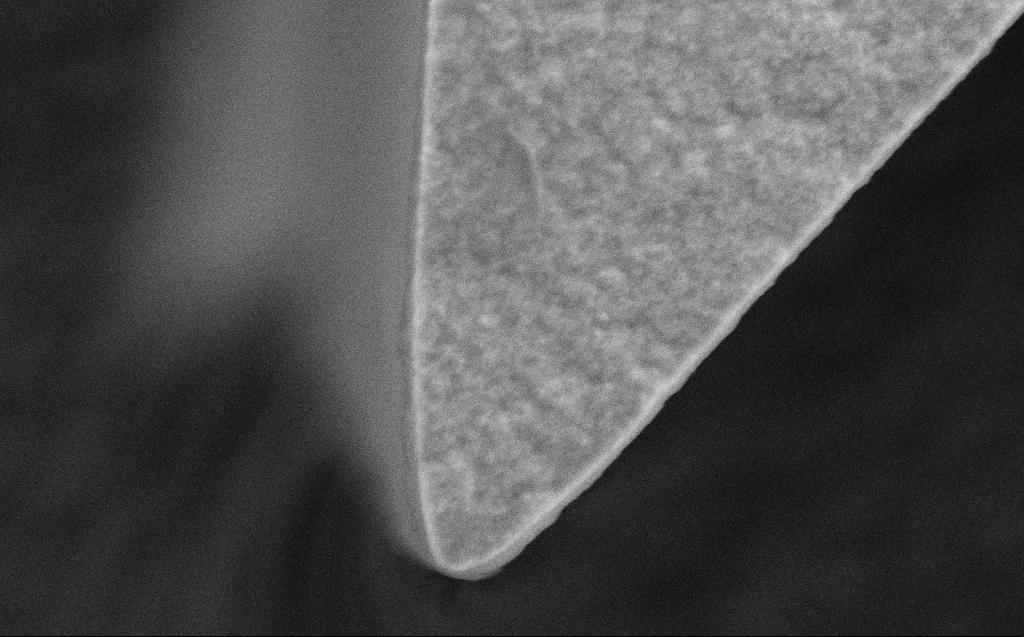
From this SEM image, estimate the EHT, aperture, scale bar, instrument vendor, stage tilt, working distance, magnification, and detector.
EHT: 5 kV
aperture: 30 µm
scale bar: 1000 nm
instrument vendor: Zeiss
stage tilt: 0°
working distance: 5 mm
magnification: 56.65 K X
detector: SE2